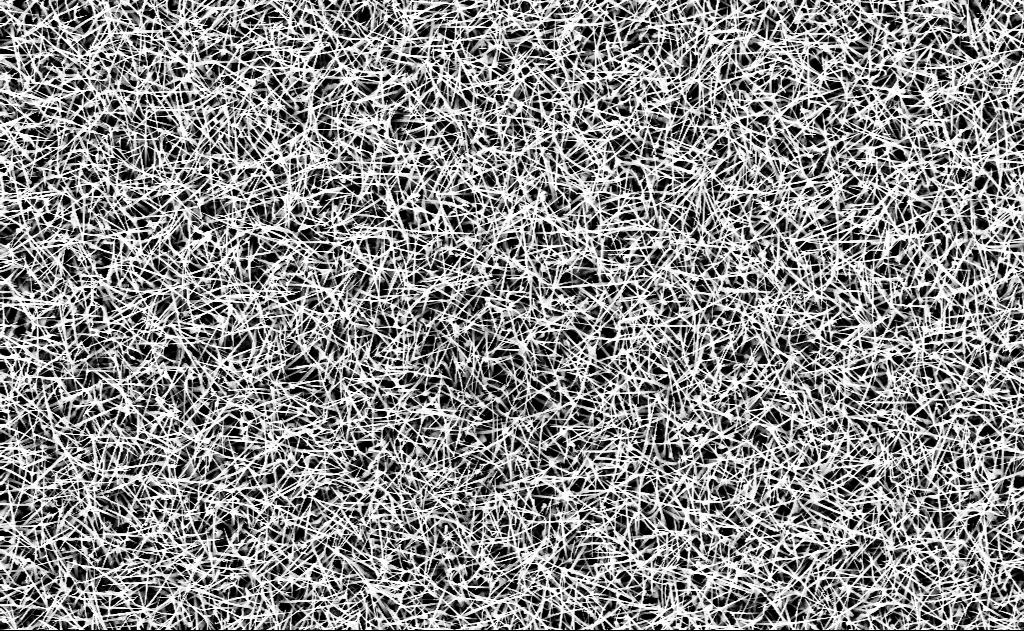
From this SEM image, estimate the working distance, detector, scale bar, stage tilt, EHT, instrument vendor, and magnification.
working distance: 13 mm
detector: InLens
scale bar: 2000 nm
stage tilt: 0°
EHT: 10 kV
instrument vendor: Zeiss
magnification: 10 K X